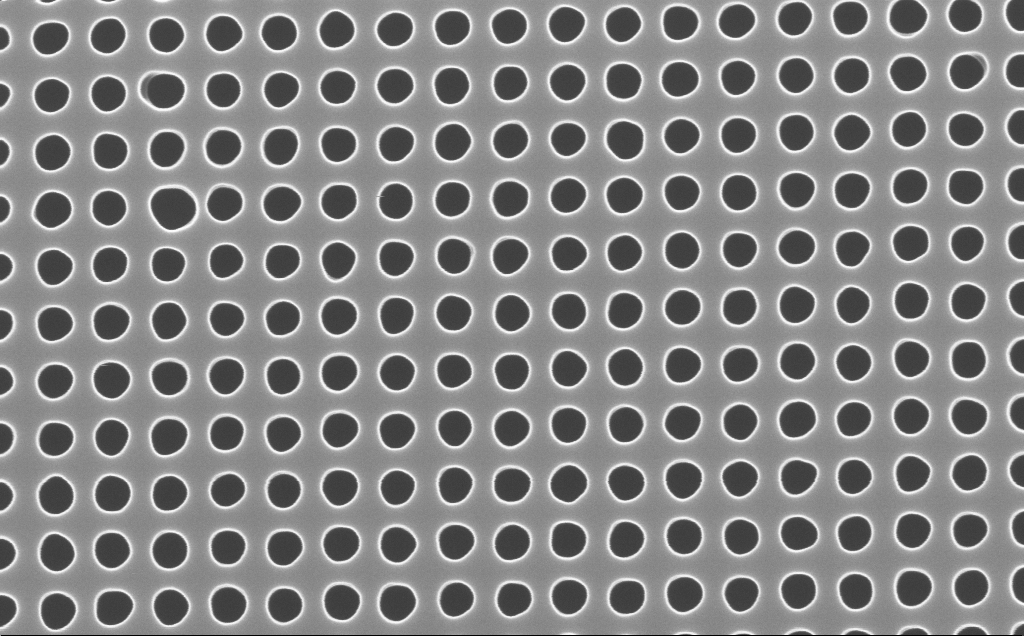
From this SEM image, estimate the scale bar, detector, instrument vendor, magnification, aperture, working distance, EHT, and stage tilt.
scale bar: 100 nm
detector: InLens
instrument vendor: Zeiss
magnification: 140 K X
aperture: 30 µm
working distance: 4 mm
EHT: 10 kV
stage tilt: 0°